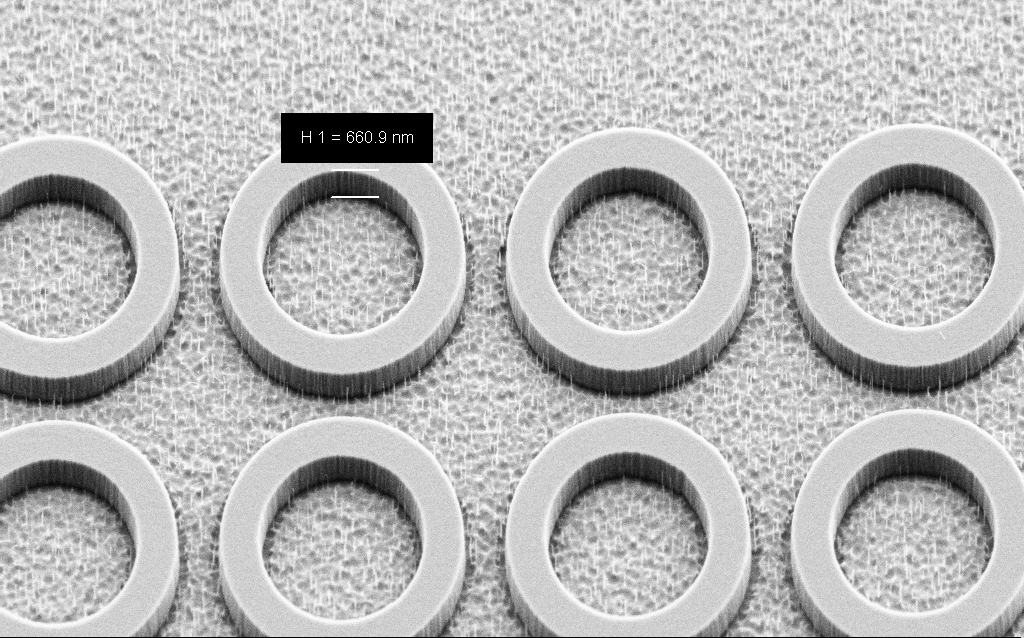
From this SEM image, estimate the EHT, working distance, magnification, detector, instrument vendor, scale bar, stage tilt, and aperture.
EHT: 3 kV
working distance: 8 mm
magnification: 15 K X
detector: SE2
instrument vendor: Zeiss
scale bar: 1000 nm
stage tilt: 45°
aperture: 30 µm